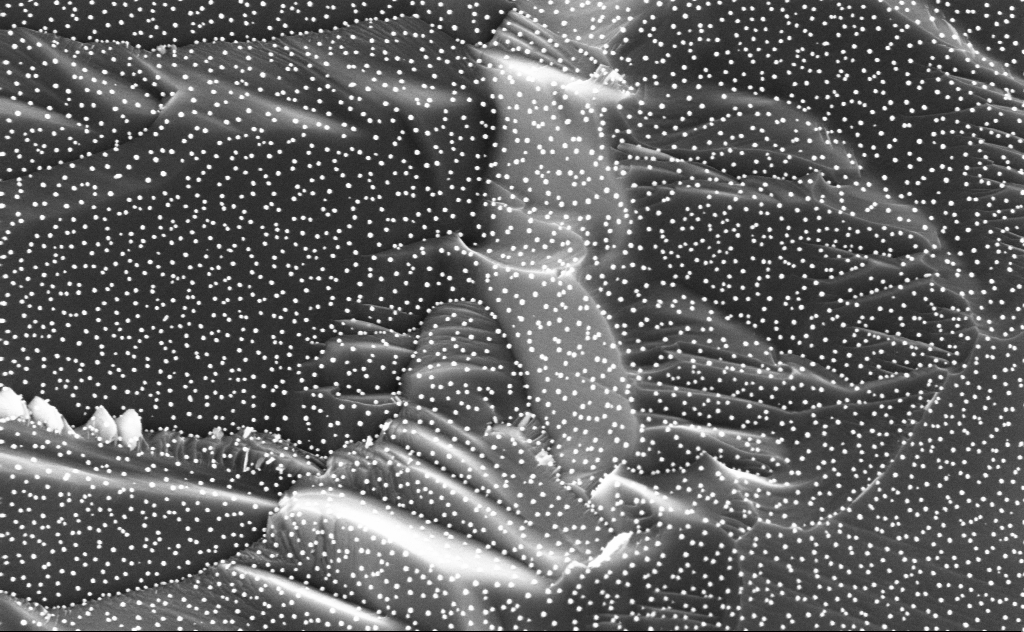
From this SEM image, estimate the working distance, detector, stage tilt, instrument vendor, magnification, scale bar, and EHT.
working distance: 5 mm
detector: InLens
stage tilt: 0°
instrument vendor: Zeiss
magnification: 80 K X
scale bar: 200 nm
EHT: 3 kV